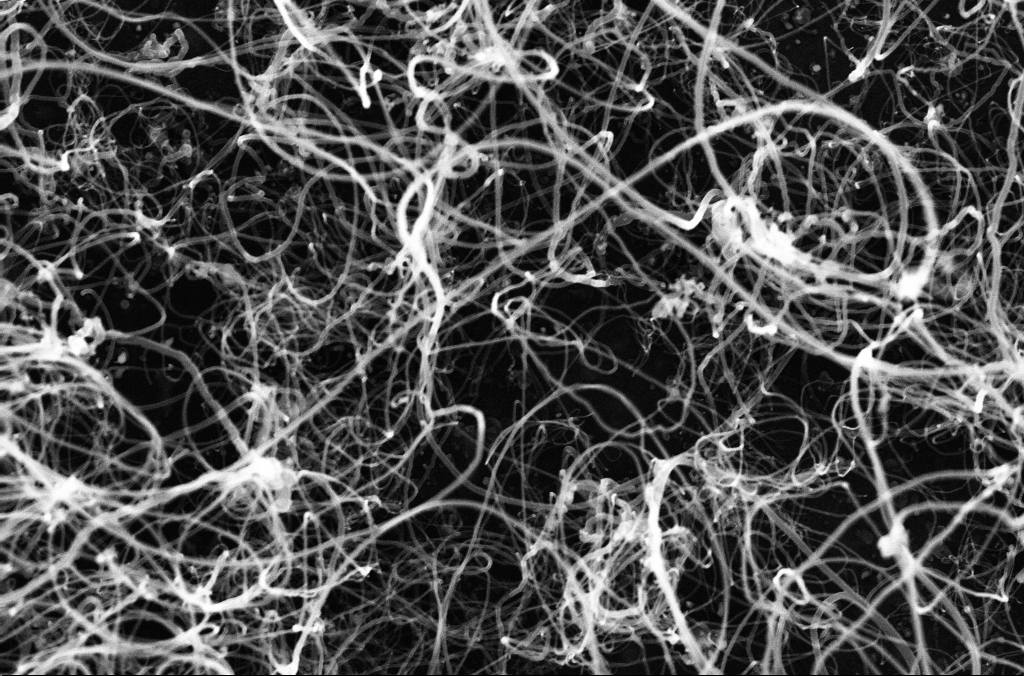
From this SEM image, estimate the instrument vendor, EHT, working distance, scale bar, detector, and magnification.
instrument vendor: Zeiss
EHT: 10 kV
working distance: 3.3 mm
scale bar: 1000 nm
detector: InLens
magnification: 50 K X